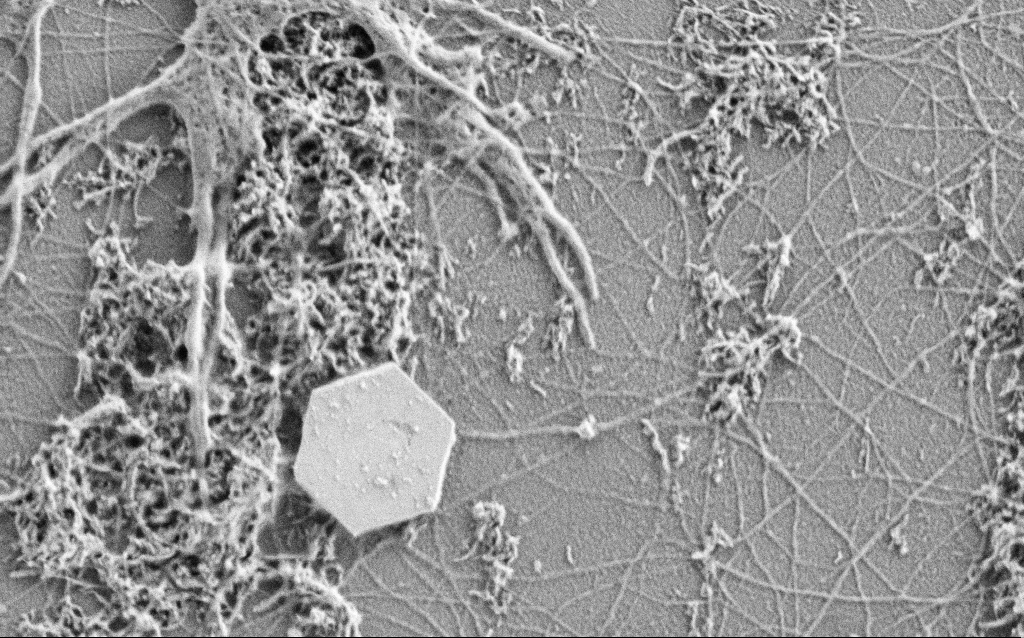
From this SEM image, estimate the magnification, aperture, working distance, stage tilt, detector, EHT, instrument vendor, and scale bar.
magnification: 33 K X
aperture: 30 µm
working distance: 4 mm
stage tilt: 0°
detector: SE2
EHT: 1 kV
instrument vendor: Zeiss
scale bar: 2000 nm